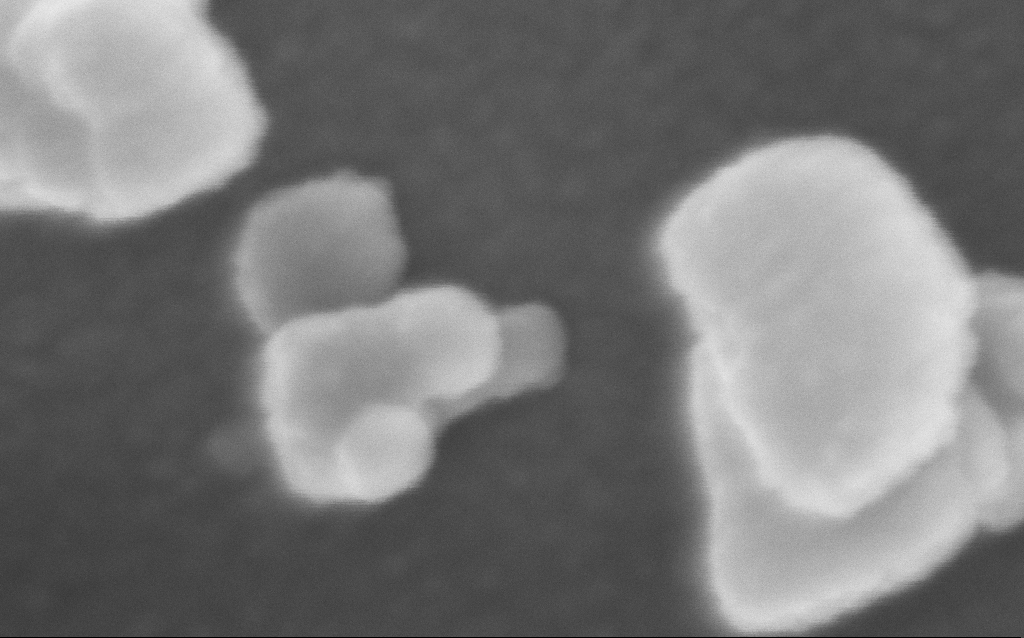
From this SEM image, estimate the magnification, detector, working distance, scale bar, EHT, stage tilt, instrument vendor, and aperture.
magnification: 603.72 K X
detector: InLens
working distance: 6 mm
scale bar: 100 nm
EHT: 10 kV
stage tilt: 0°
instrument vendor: Zeiss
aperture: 30 µm